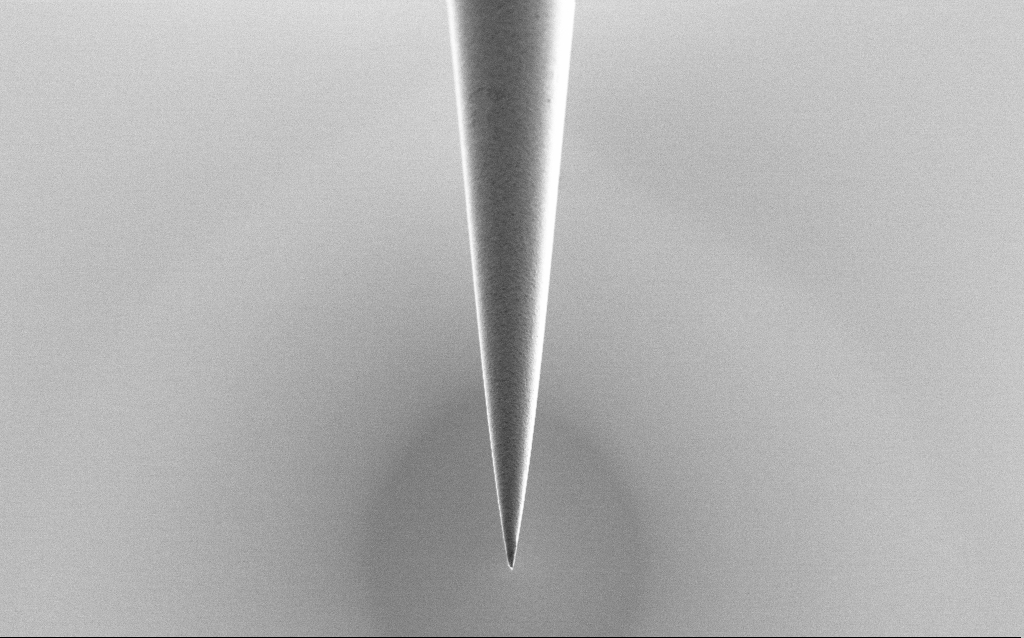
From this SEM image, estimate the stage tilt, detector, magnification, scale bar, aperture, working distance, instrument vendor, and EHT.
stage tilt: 45°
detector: SE2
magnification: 10 K X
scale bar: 2000 nm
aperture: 30 µm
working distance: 6 mm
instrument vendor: Zeiss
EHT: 1 kV